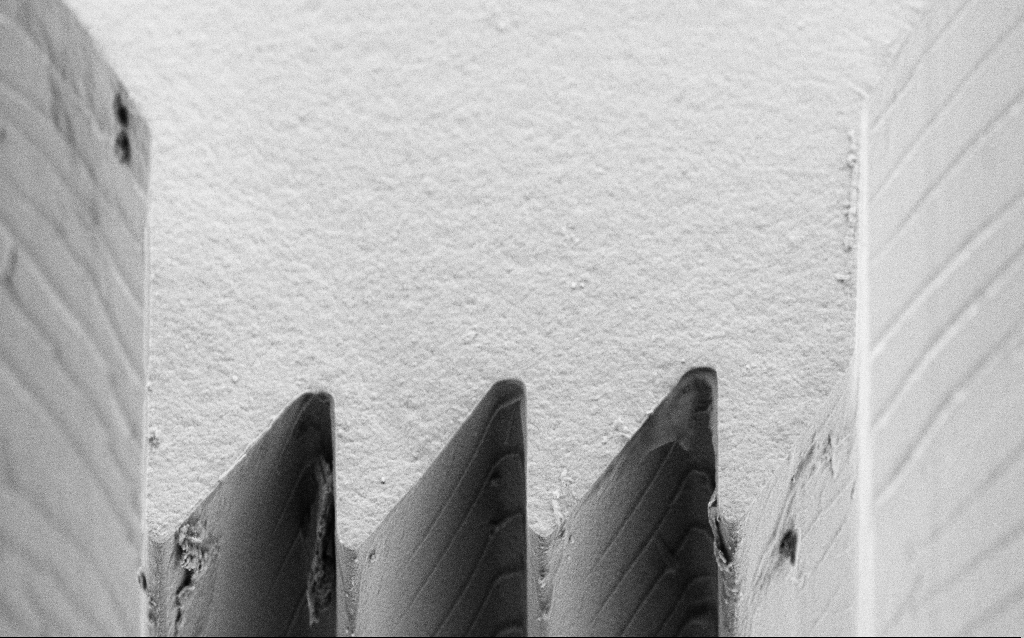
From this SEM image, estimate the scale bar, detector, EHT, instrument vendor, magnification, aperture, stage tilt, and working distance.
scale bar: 10000 nm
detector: SE2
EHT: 5 kV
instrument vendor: Zeiss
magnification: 6.25 K X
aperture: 30 µm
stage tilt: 45°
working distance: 6 mm